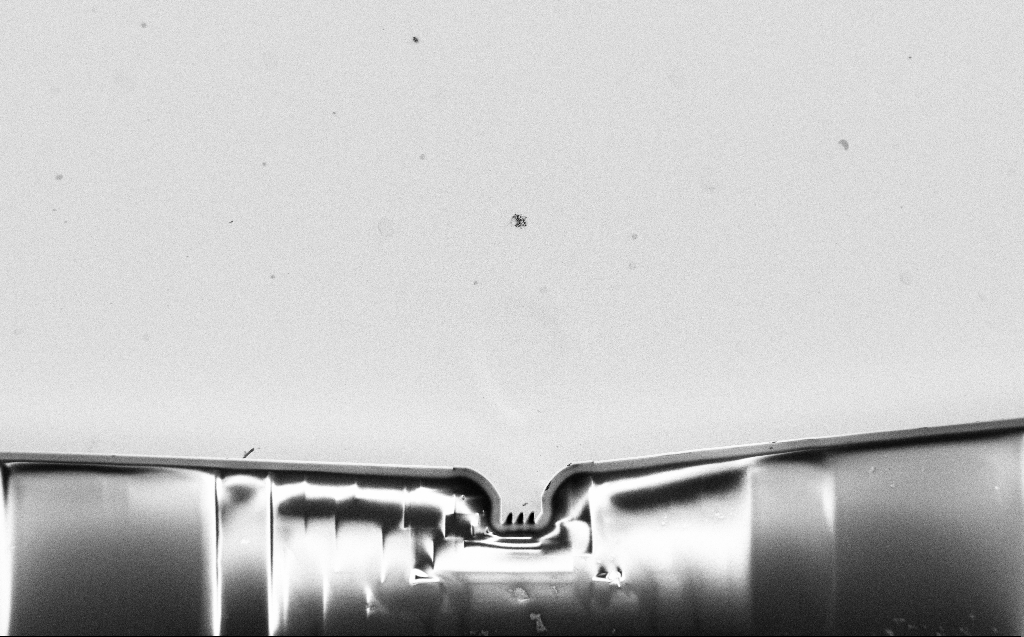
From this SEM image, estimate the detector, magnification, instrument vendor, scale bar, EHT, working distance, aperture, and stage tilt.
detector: SE2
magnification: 0.346 K X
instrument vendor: Zeiss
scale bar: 100000 nm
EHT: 2 kV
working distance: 9 mm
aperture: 30 µm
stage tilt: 0°